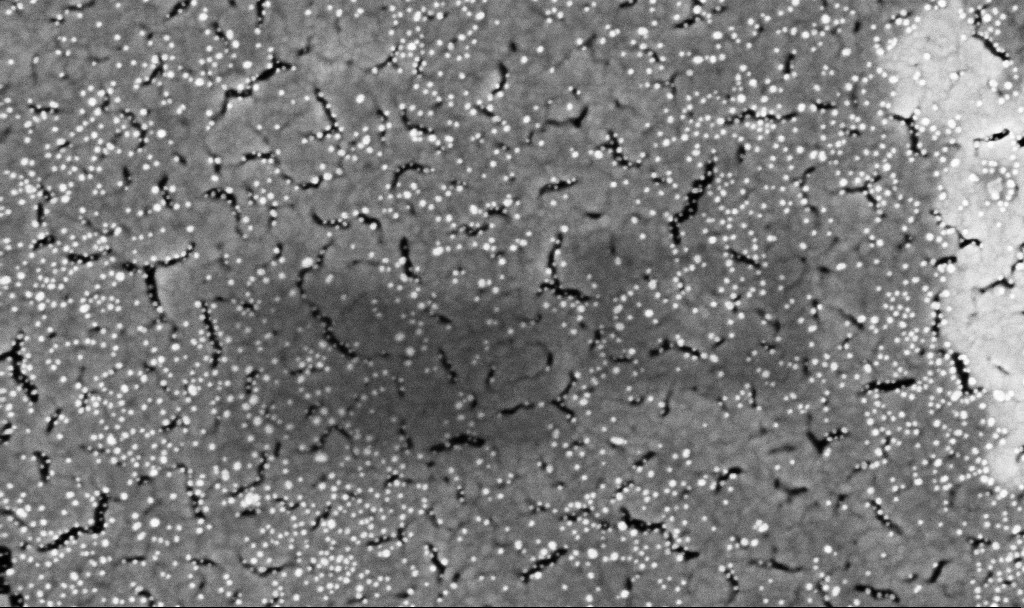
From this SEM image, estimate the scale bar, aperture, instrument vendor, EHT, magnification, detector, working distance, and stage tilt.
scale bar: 200 nm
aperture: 30 µm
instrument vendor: Zeiss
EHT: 5 kV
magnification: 107.34 K X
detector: InLens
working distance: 3.3 mm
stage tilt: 0°